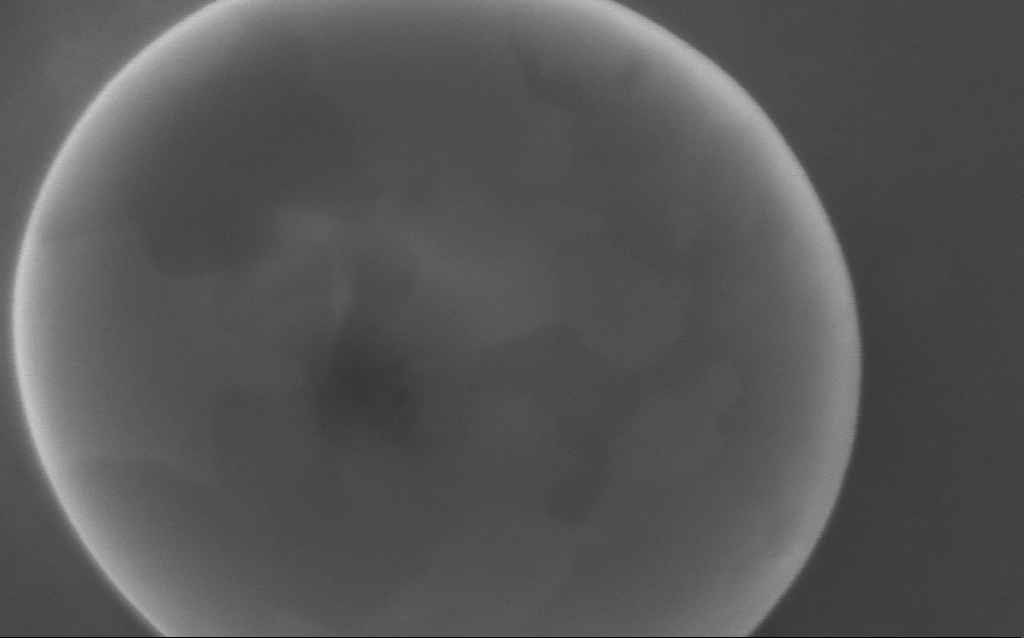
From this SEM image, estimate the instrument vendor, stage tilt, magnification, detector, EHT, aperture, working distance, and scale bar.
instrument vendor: Zeiss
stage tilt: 0°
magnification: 179.94 K X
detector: InLens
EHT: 5 kV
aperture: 30 µm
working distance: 3 mm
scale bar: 100 nm